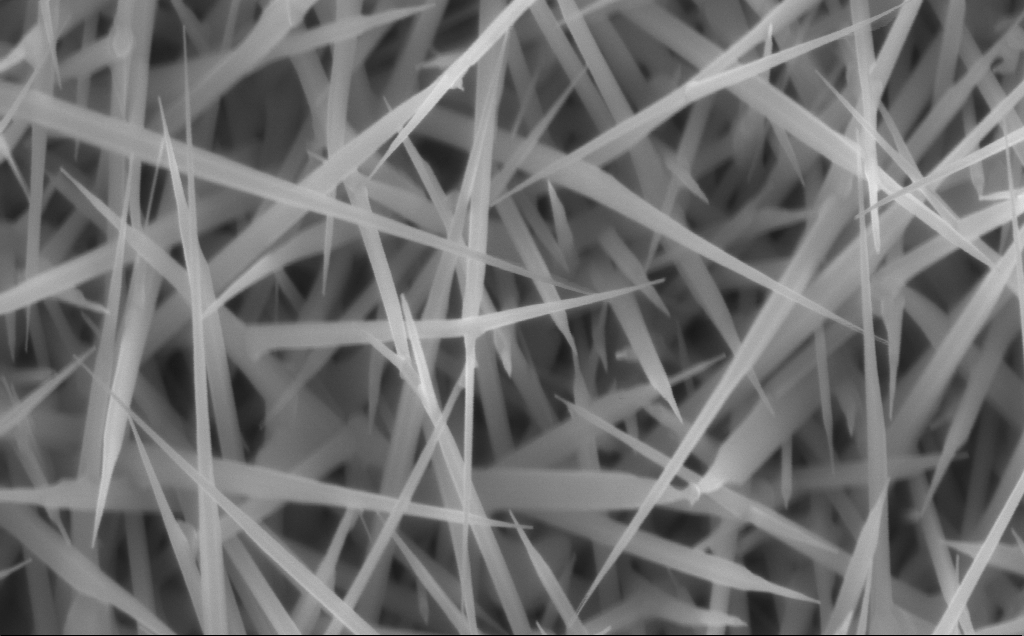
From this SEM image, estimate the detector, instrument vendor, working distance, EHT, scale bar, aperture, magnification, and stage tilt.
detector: InLens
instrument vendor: Zeiss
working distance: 7 mm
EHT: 10 kV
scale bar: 200 nm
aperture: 30 µm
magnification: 80 K X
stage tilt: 0°